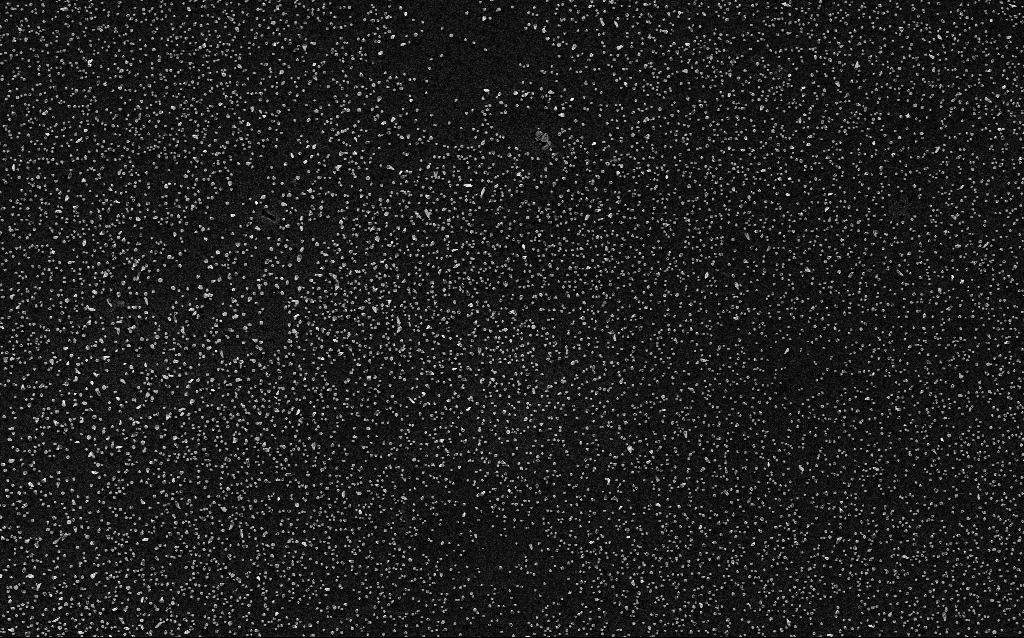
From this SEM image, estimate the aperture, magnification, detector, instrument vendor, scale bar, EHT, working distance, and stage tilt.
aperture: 30 µm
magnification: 10 K X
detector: InLens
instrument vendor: Zeiss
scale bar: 2000 nm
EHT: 5 kV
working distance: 1.9 mm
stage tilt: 0°